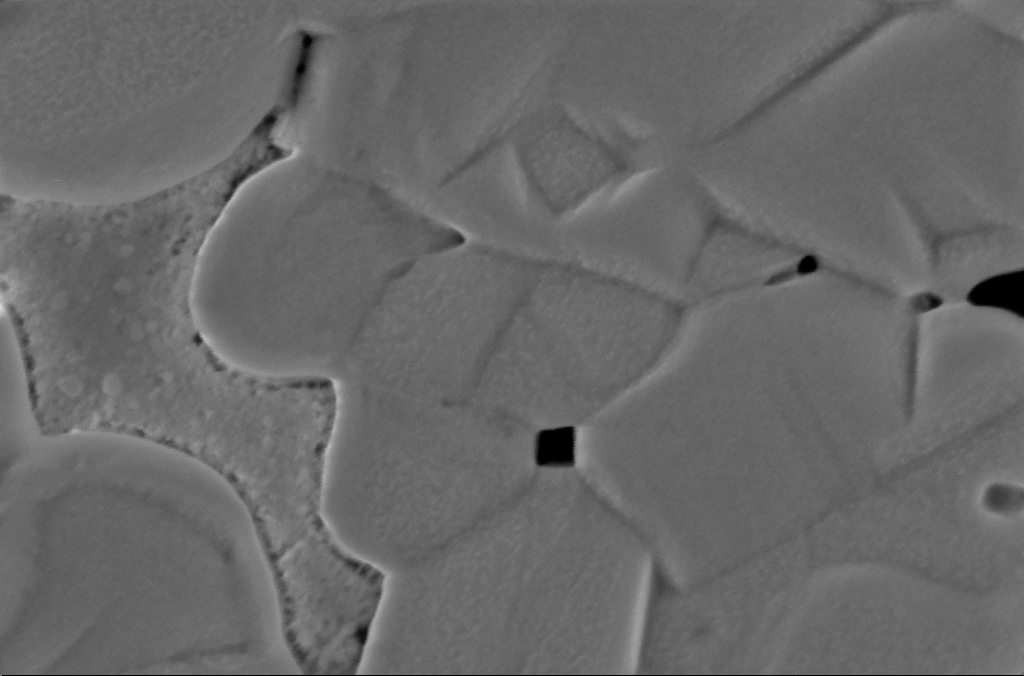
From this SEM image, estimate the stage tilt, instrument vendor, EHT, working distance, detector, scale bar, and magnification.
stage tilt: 0°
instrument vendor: Zeiss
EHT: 2 kV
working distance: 3 mm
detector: InLens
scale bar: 200 nm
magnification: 205.62 K X